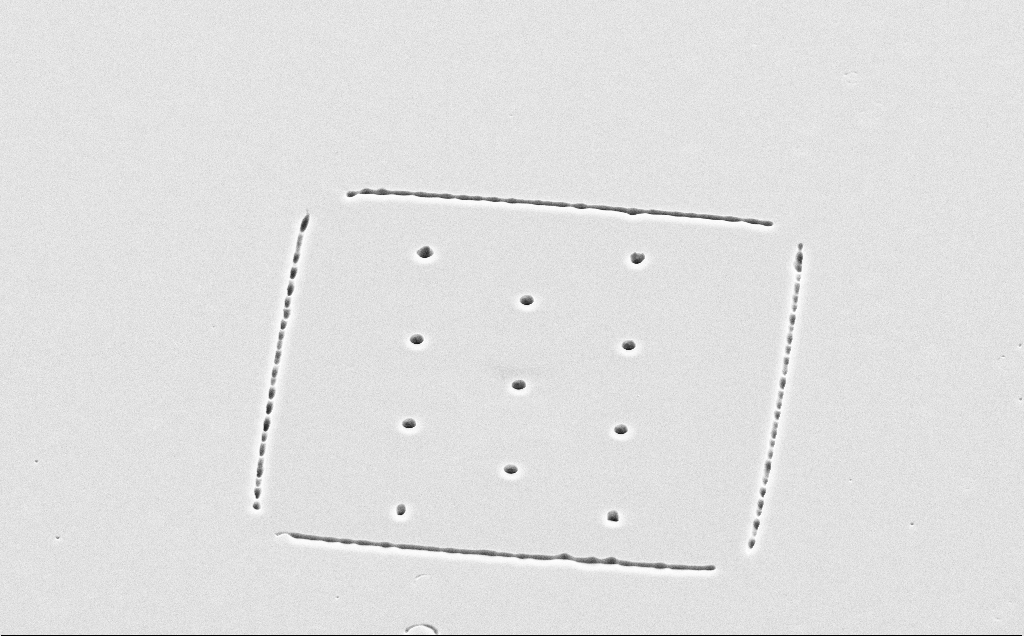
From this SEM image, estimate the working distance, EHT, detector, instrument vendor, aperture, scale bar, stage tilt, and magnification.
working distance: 13 mm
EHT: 10 kV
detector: SE2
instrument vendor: Zeiss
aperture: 30 µm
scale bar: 20000 nm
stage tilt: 45°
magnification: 2.2 K X